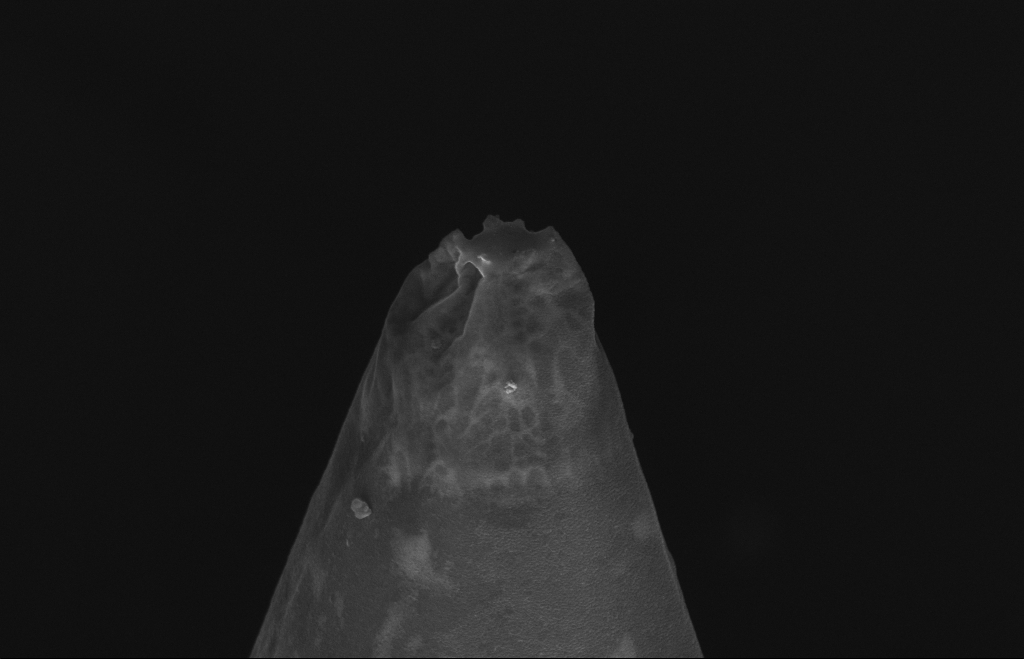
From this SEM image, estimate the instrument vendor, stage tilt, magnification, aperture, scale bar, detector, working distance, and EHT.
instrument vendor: Zeiss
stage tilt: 70°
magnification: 43.78 K X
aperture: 30 µm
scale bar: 1000 nm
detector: InLens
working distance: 8 mm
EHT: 10 kV